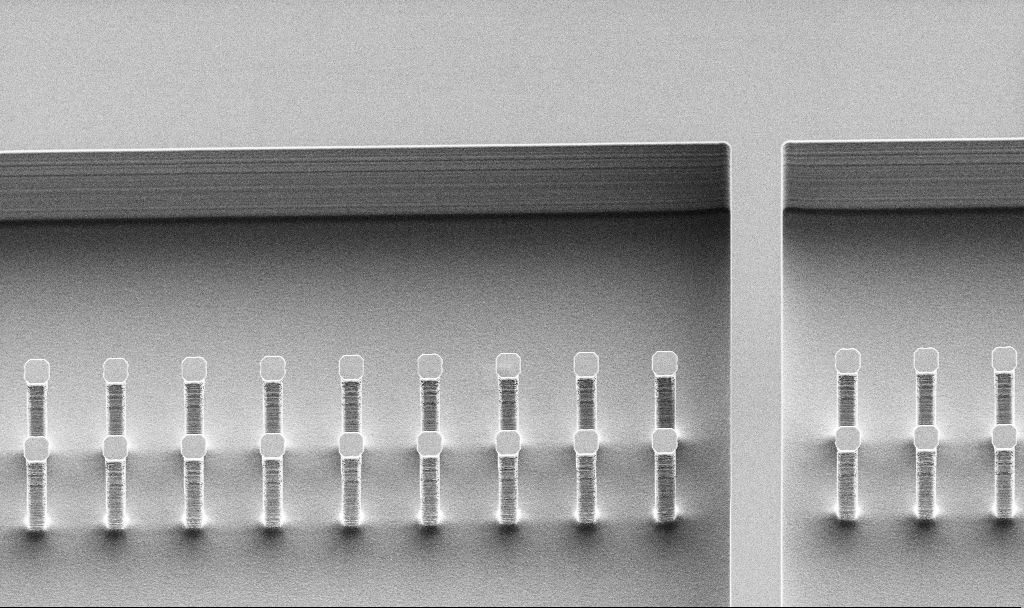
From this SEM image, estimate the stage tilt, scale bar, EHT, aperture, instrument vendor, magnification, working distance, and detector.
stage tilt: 45°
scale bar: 20000 nm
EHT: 5 kV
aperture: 30 µm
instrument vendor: Zeiss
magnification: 0.955 K X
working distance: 13.7 mm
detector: SE2